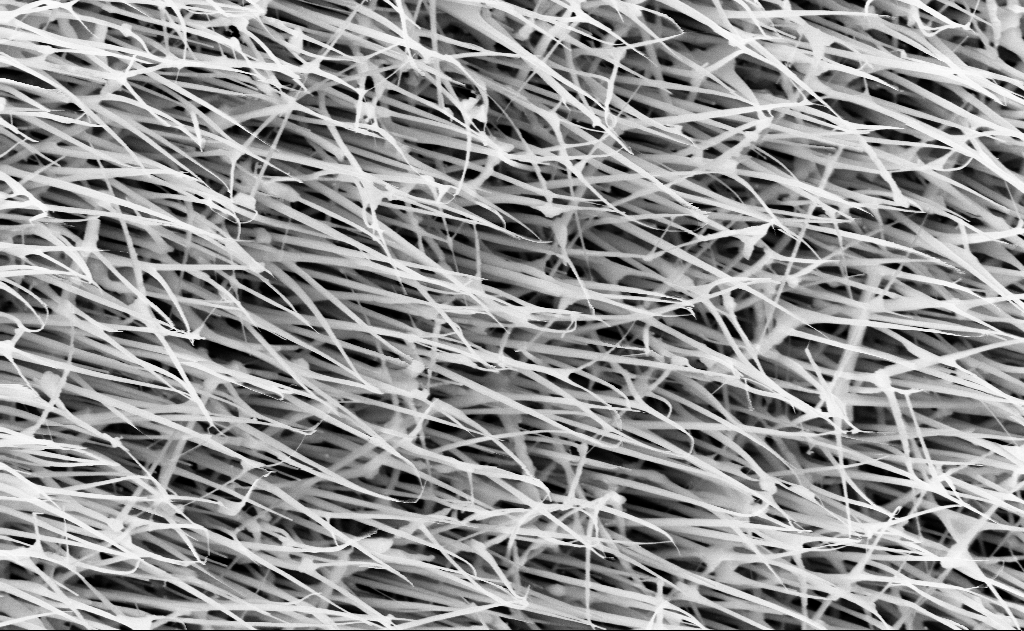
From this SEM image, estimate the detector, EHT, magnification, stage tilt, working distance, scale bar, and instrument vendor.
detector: InLens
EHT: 10 kV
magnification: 40 K X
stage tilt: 0°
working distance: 13 mm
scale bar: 1000 nm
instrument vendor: Zeiss